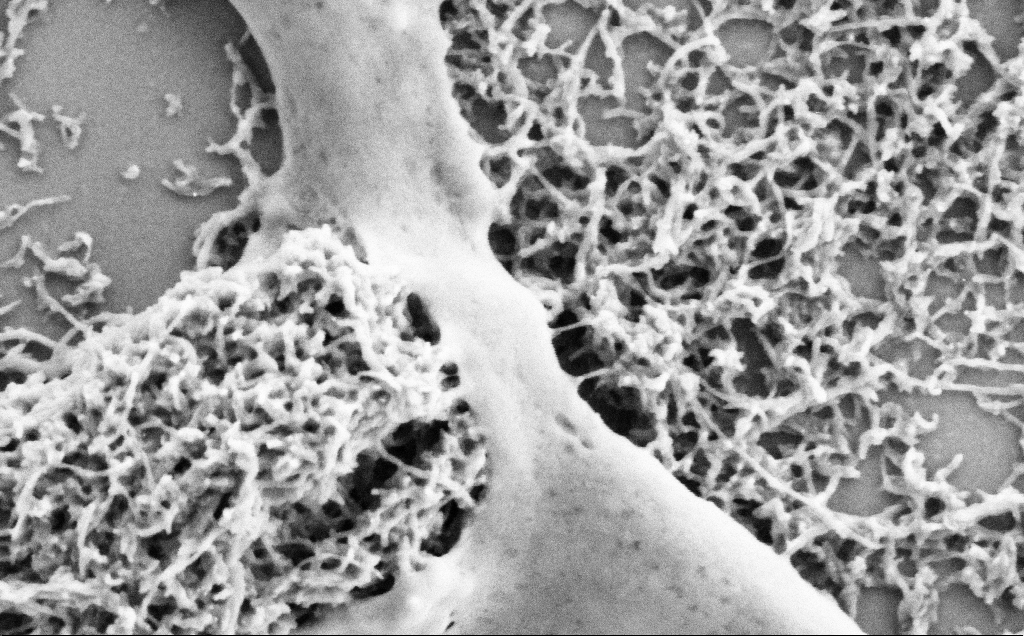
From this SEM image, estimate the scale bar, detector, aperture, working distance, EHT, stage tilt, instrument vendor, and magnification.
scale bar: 200 nm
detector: SE2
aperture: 30 µm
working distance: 7.1 mm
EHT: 2 kV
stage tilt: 0°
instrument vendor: Zeiss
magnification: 75 K X